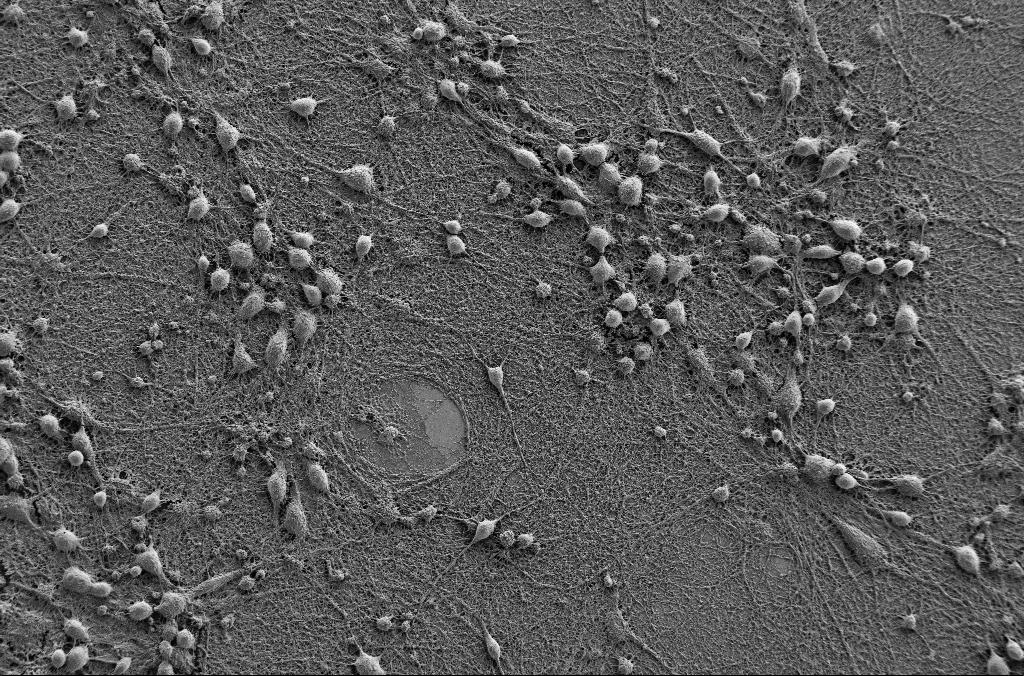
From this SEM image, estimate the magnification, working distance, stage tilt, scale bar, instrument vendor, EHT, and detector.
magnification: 1 K X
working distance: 4.1 mm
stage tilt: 0°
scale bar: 20000 nm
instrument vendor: Zeiss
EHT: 1 kV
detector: SE2